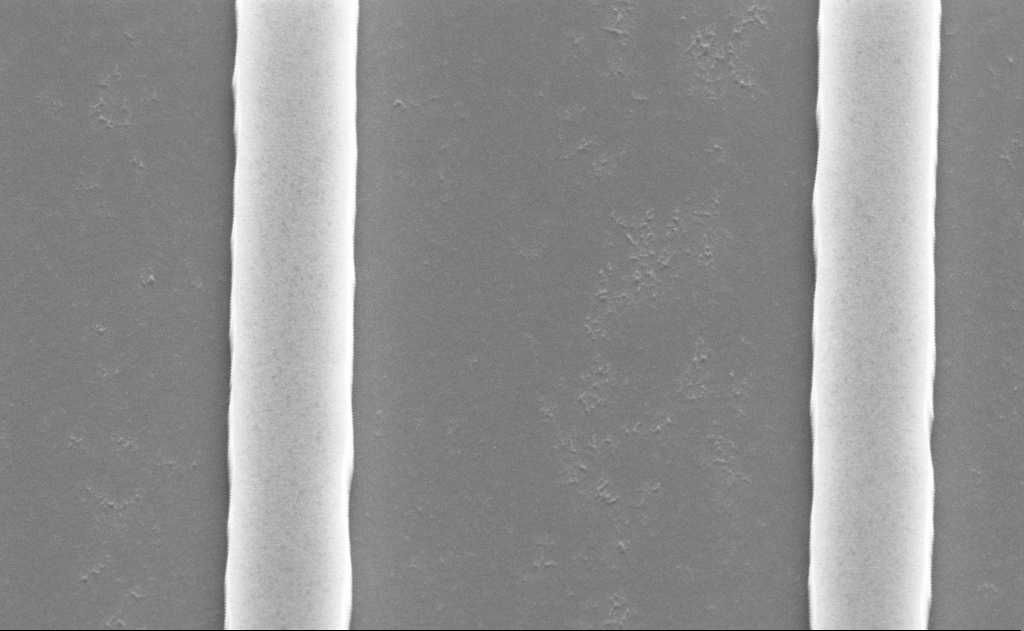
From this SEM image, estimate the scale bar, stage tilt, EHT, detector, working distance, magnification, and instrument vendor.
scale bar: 1000 nm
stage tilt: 55°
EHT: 5 kV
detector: SE2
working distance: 13 mm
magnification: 53.23 K X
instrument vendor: Zeiss